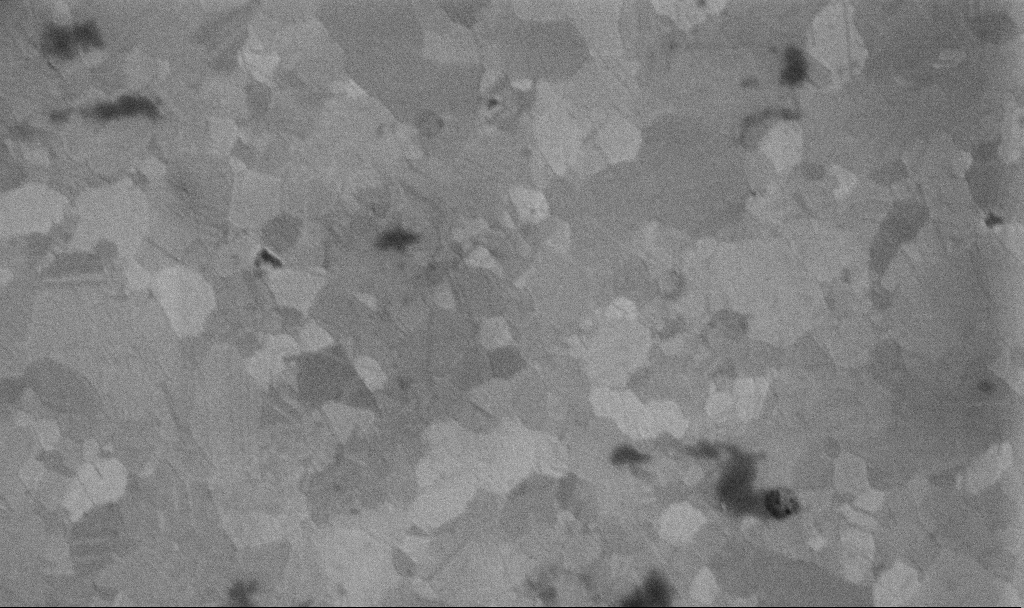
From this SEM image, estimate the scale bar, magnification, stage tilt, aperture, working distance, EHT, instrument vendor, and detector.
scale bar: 200 nm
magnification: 99.87 K X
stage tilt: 0°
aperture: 30 µm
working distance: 3.3 mm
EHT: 10 kV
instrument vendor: Zeiss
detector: InLens